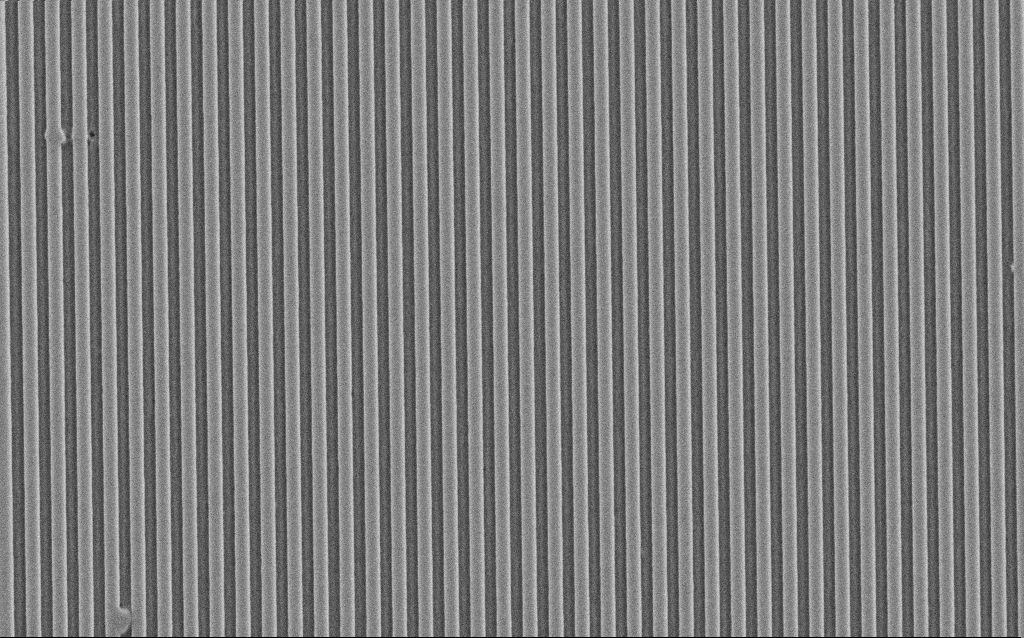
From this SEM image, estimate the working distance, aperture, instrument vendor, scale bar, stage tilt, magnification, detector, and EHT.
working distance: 5 mm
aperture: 30 µm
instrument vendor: Zeiss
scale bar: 1000 nm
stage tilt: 0°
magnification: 19.34 K X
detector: SE2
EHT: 3 kV